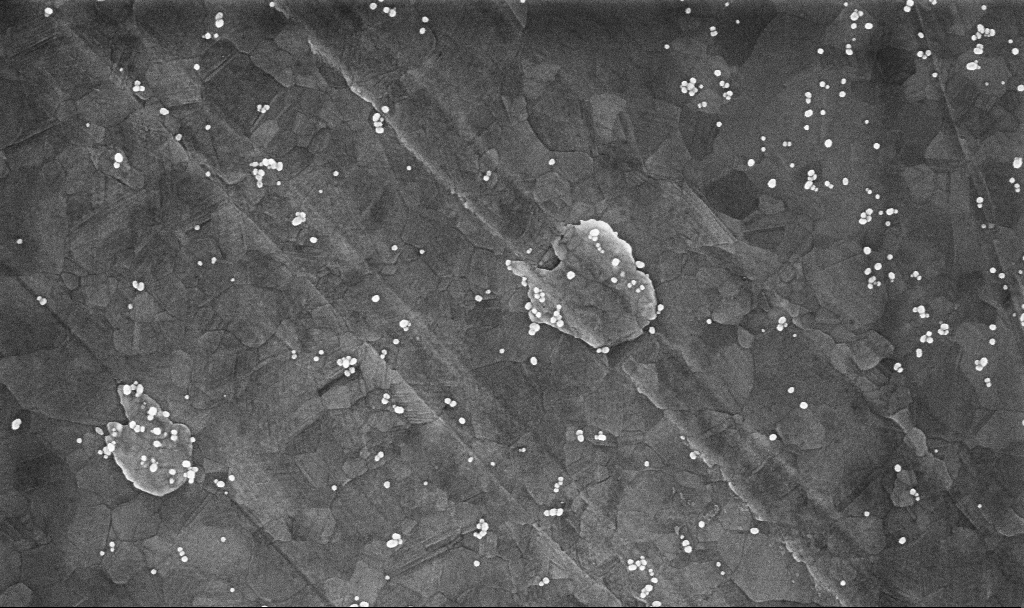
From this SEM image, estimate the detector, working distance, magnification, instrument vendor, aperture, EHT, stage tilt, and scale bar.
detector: InLens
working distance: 3.4 mm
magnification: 100.42 K X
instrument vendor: Zeiss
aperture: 30 µm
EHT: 10 kV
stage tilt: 0°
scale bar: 200 nm